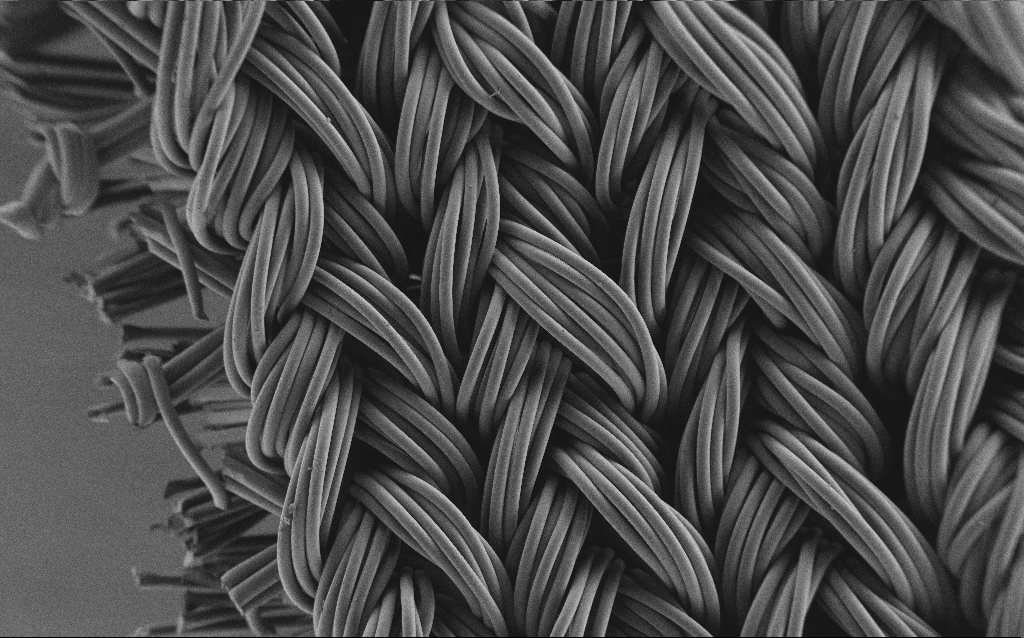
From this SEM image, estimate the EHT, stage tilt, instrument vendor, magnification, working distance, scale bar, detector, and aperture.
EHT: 1 kV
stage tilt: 0°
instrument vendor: Zeiss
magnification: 0.199 K X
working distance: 4 mm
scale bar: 100000 nm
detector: SE2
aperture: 30 µm